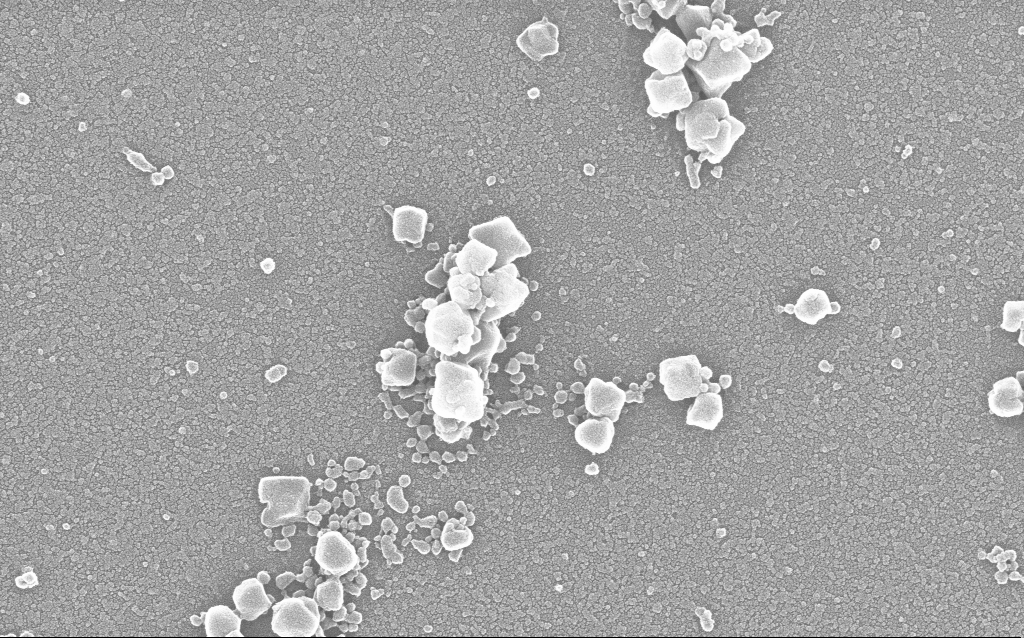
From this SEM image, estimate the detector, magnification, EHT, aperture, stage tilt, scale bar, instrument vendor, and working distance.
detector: InLens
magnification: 100 K X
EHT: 20 kV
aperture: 30 µm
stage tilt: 0°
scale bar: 200 nm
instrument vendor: Zeiss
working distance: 1.9 mm